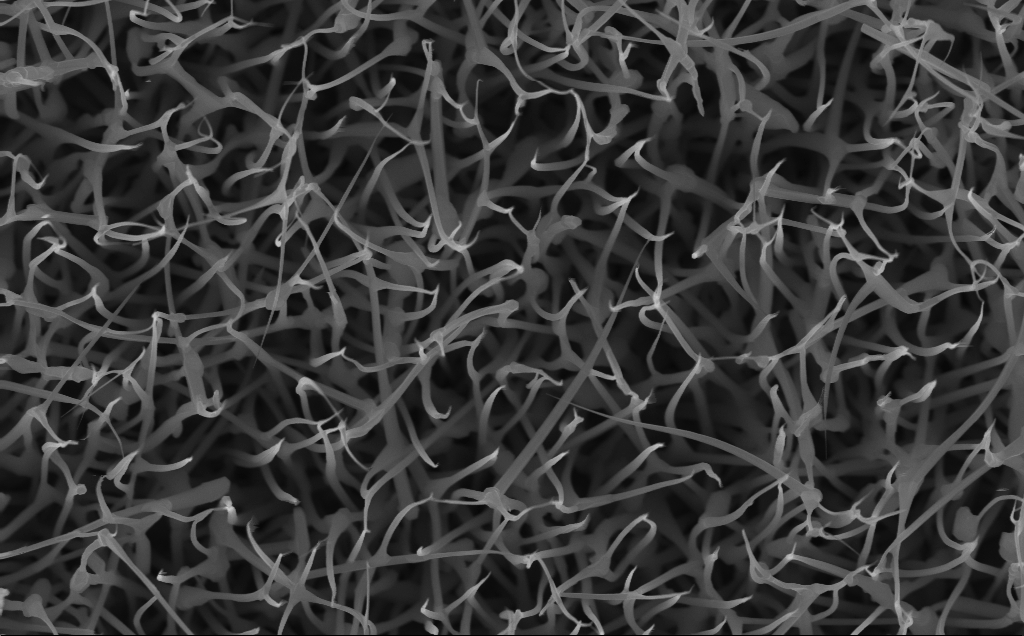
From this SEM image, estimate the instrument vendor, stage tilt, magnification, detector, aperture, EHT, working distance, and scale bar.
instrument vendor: Zeiss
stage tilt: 0°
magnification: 40 K X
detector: InLens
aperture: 30 µm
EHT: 10 kV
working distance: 4 mm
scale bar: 1000 nm